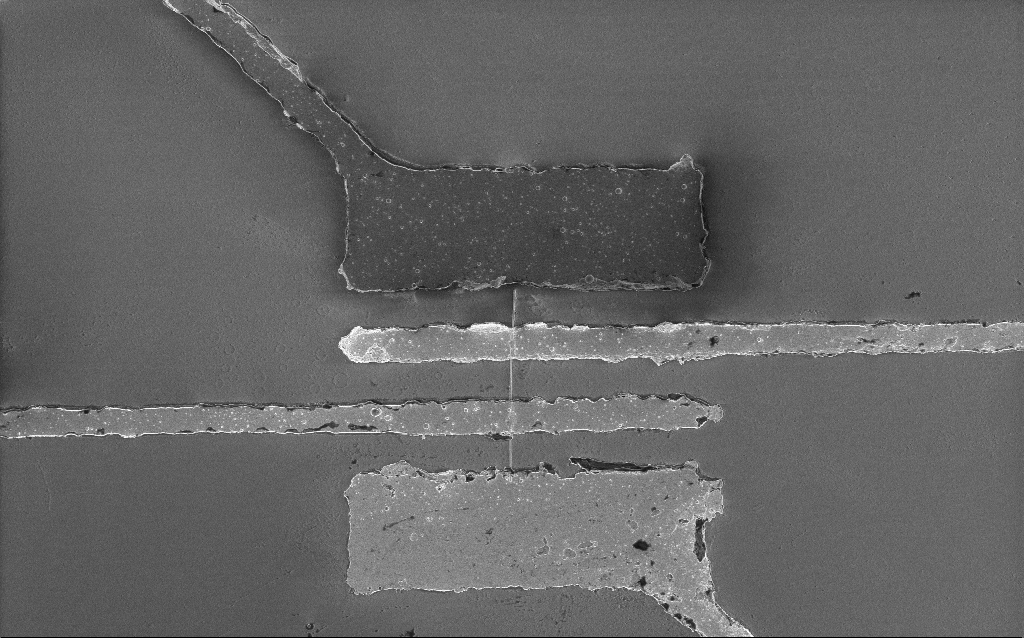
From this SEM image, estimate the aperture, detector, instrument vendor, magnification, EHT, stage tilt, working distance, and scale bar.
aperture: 30 µm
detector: InLens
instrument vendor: Zeiss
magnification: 4.51 K X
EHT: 2 kV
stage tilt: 0°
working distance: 7.5 mm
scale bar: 10000 nm